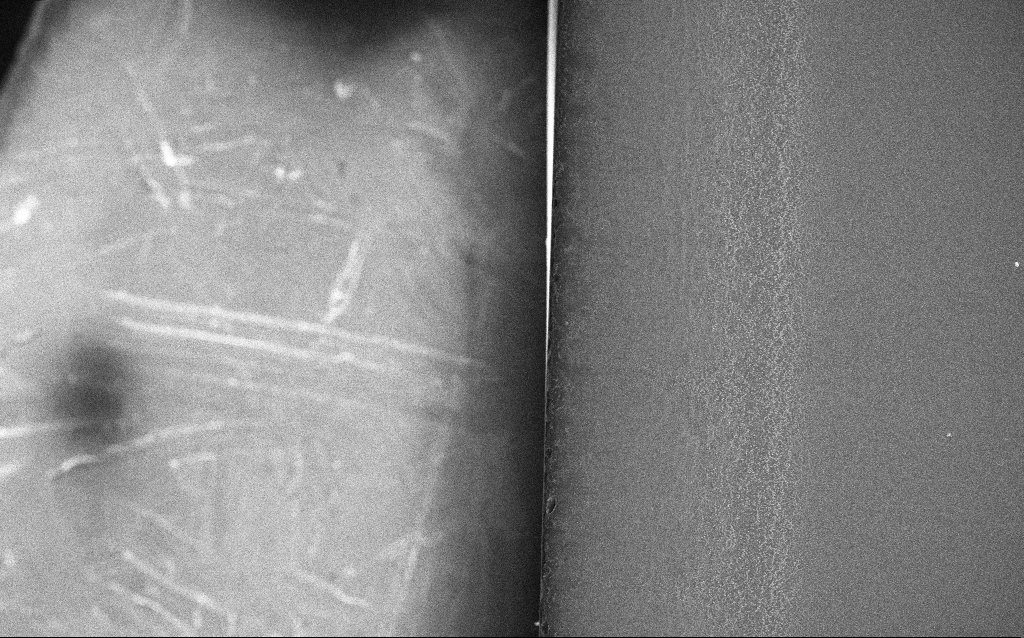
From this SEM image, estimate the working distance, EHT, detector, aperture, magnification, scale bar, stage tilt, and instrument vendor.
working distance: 1.4 mm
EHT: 20 kV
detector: InLens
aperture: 30 µm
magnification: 0.25 K X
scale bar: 100000 nm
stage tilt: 0°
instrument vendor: Zeiss